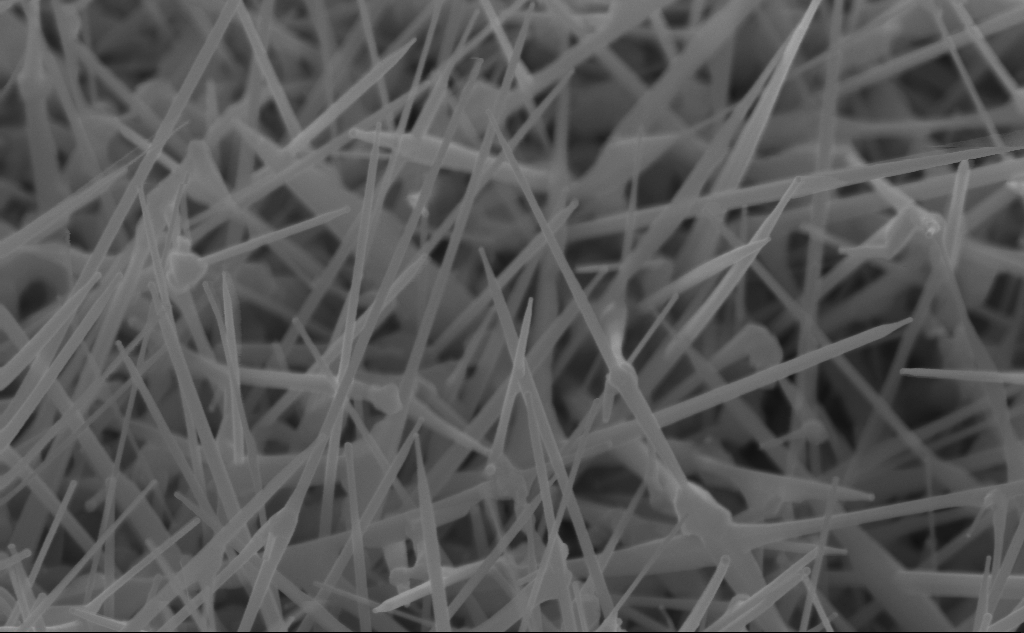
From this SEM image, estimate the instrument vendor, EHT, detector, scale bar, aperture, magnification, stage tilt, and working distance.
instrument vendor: Zeiss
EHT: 10 kV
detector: InLens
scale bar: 200 nm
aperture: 30 µm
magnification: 80 K X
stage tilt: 45°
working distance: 4 mm